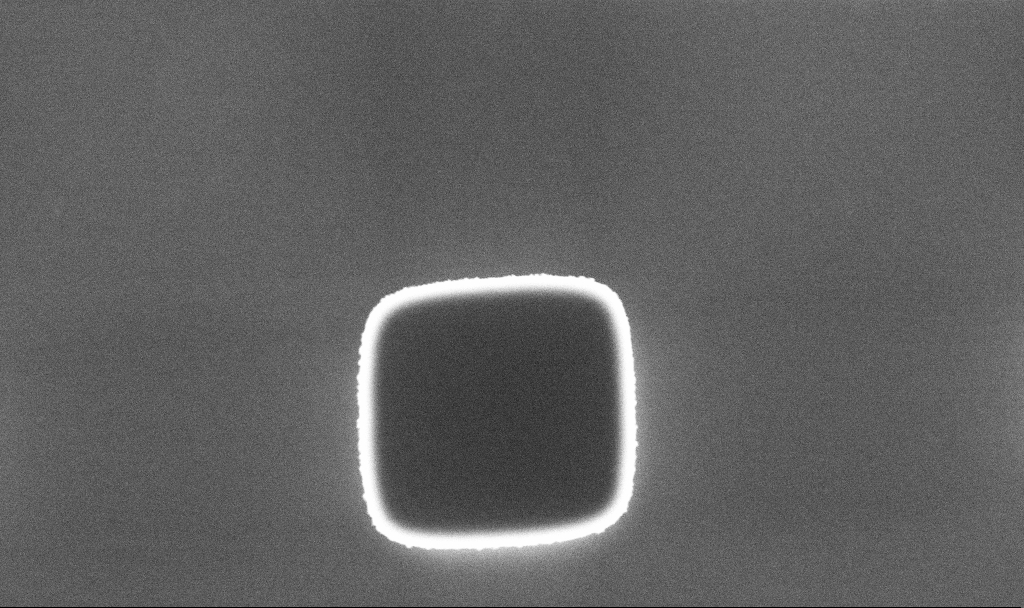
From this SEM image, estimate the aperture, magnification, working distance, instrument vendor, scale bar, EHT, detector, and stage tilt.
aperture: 30 µm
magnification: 20.96 K X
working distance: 5.1 mm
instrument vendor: Zeiss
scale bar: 2000 nm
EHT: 5 kV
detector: InLens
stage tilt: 0°